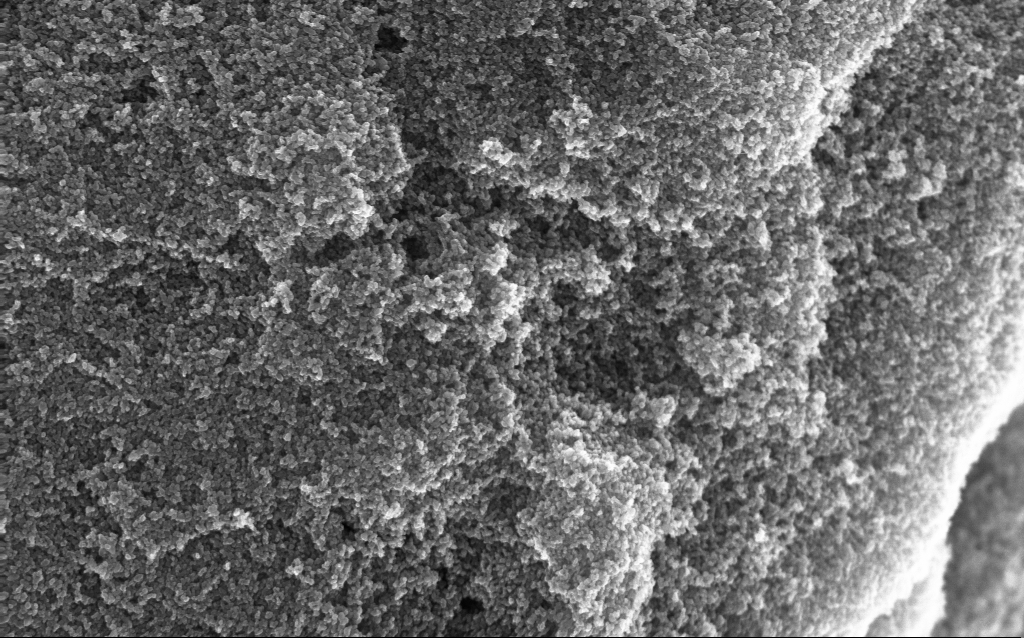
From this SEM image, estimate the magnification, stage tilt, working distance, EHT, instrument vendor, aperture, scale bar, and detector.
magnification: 65.04 K X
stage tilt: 0°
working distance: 2.8 mm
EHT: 10 kV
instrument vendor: Zeiss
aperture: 30 µm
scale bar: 1000 nm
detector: InLens